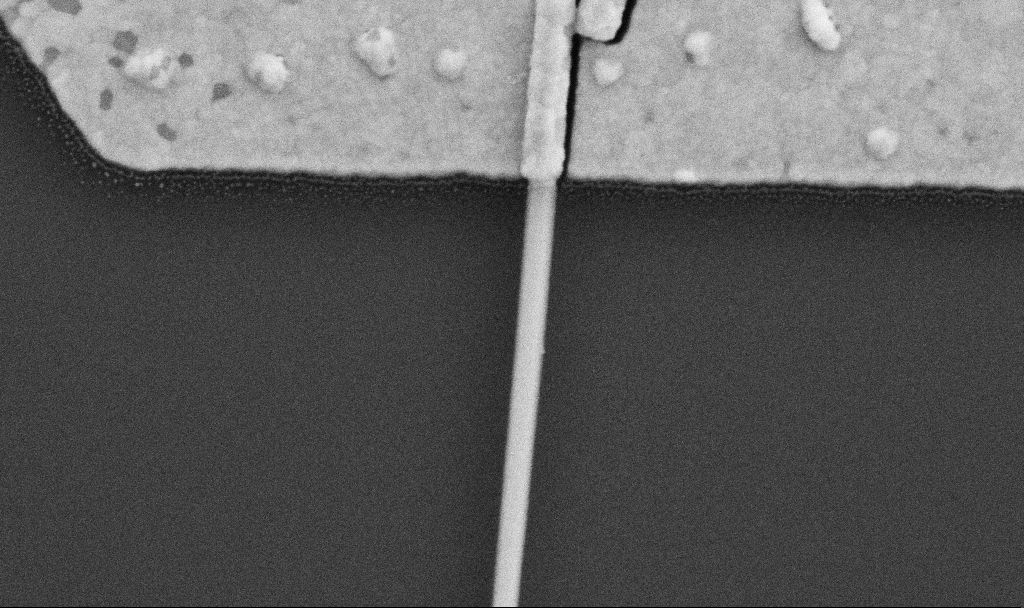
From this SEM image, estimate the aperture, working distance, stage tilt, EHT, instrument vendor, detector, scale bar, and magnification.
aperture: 30 µm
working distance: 8.7 mm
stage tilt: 0°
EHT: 5 kV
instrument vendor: Zeiss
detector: SE2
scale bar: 200 nm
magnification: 100 K X